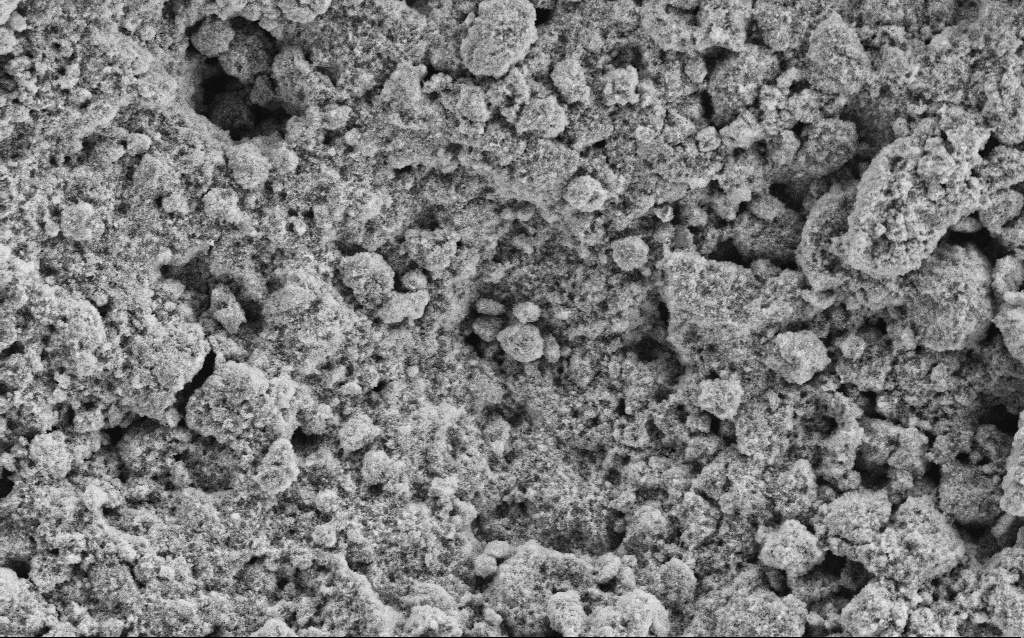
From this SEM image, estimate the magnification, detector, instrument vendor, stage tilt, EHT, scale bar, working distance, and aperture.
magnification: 6.45 K X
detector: SE2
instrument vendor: Zeiss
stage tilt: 0°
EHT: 5 kV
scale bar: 10000 nm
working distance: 4.6 mm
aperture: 30 µm